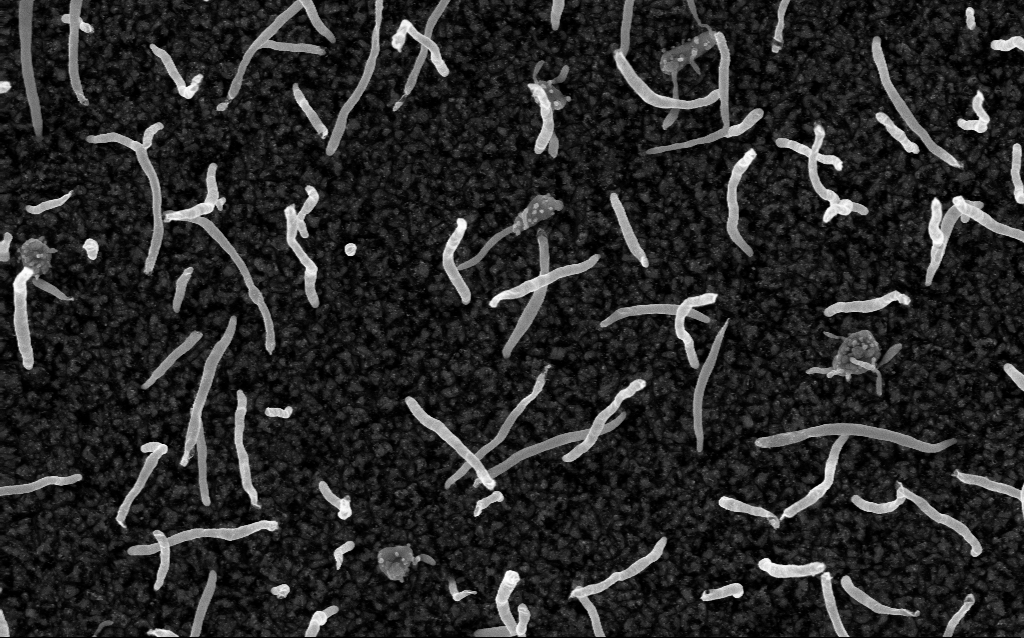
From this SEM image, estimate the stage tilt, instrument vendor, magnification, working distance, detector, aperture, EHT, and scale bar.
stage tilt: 0°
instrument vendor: Zeiss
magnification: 50 K X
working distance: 1.5 mm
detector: InLens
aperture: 30 µm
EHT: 5 kV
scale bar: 1000 nm